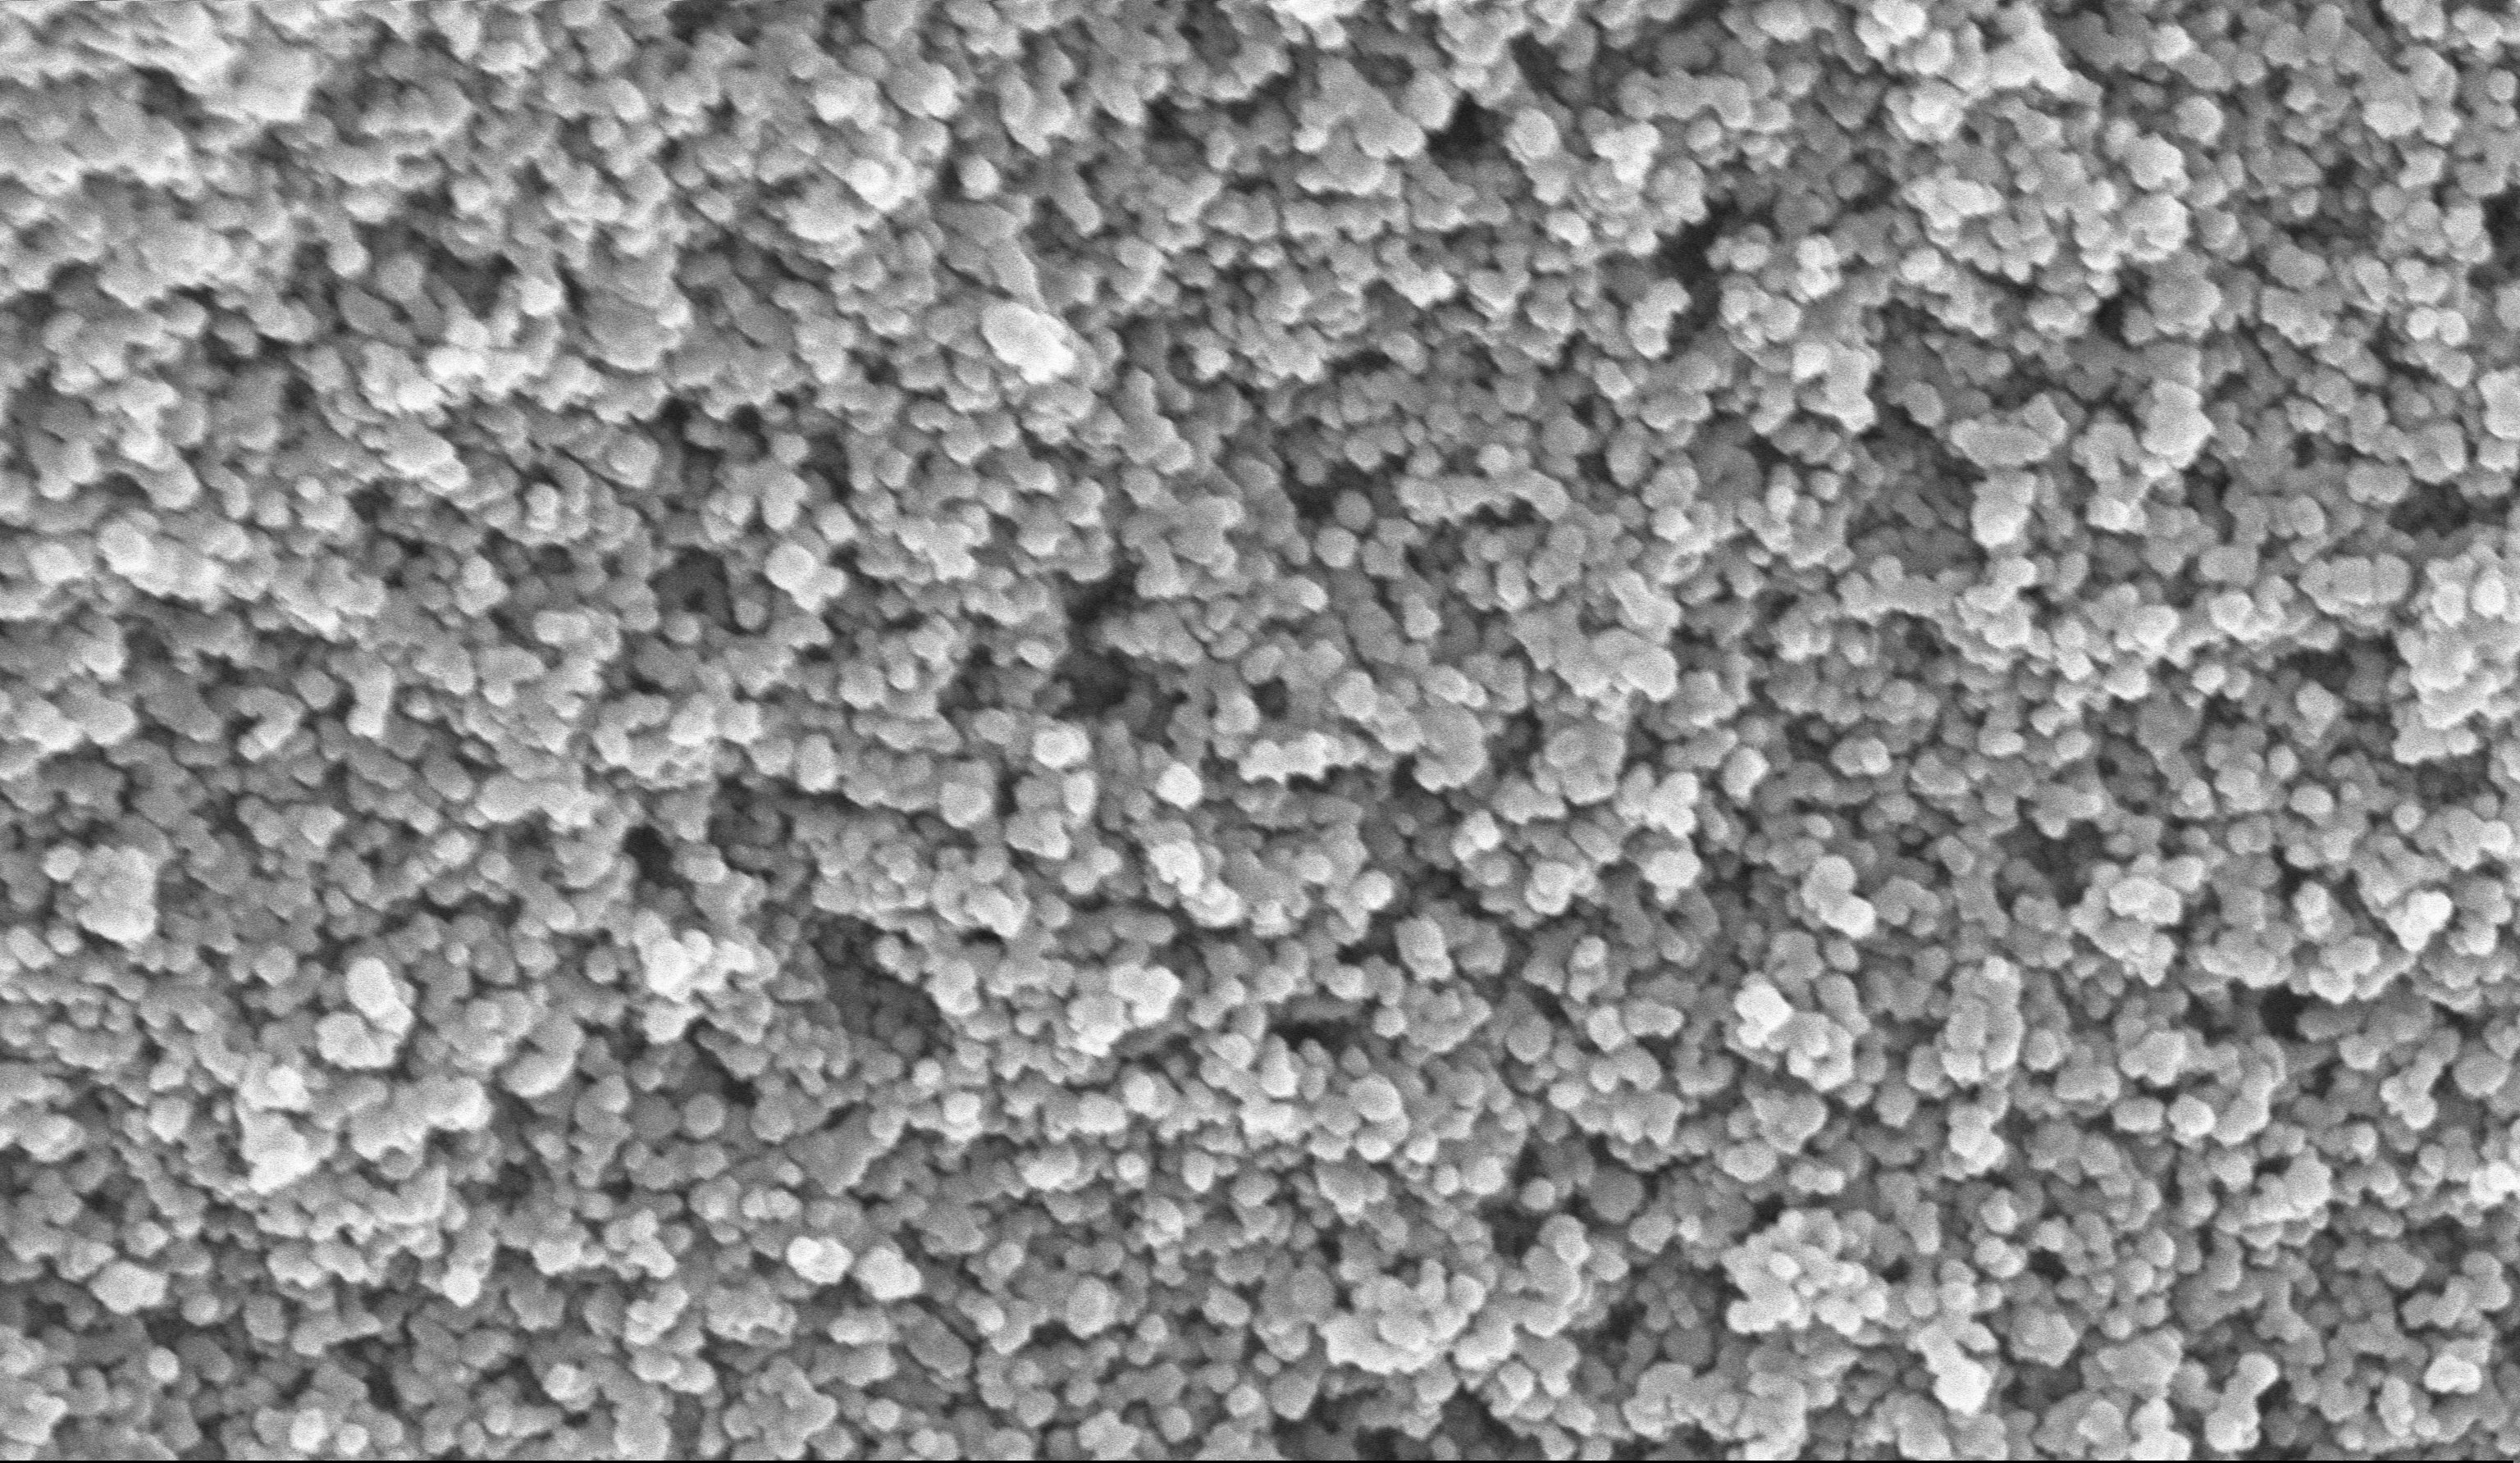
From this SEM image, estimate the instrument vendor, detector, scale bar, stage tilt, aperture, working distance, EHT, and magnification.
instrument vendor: Zeiss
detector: InLens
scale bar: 200 nm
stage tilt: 0°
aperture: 30 µm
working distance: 6 mm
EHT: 10 kV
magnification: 135 K X